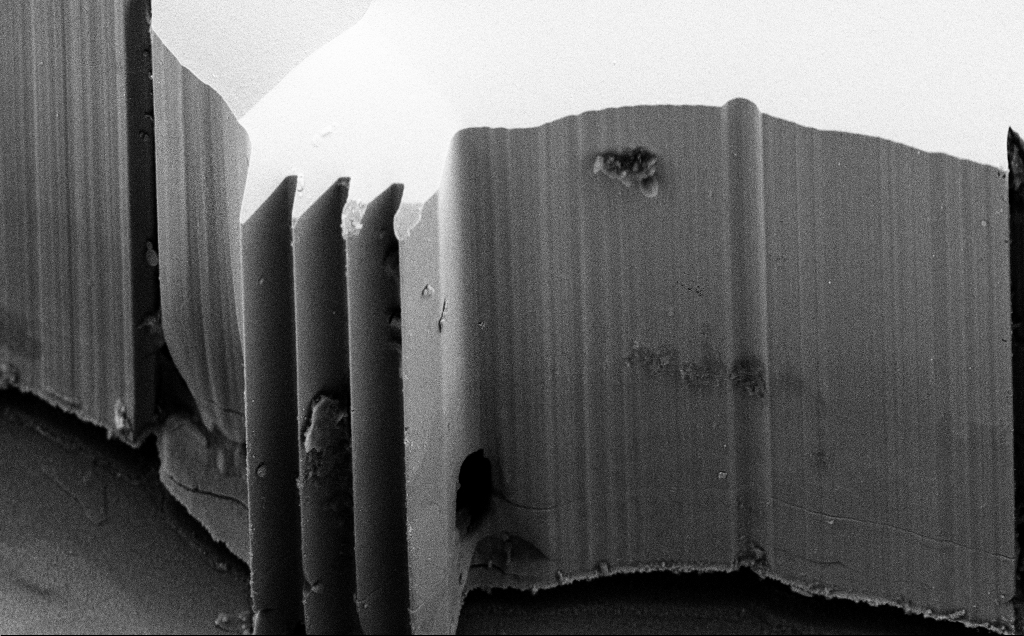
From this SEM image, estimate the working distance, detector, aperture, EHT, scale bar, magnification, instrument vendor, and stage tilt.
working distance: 8 mm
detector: SE2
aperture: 30 µm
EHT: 10 kV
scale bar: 10000 nm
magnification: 1.74 K X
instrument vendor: Zeiss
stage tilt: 45°